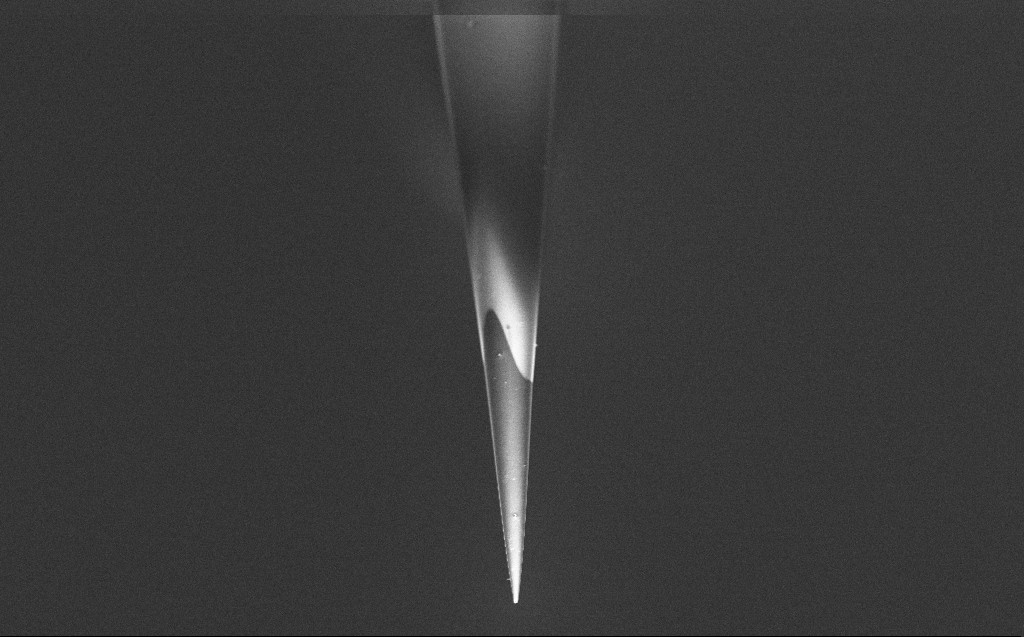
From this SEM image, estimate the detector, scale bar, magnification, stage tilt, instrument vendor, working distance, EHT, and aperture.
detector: InLens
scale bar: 10000 nm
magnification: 5 K X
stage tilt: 45°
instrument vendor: Zeiss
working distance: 6 mm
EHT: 2 kV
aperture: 30 µm